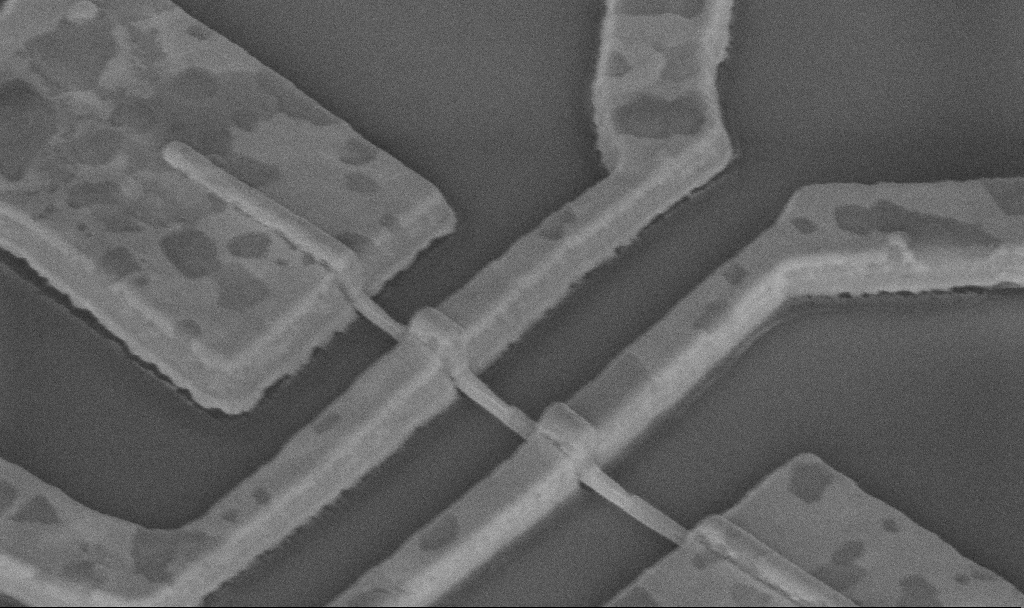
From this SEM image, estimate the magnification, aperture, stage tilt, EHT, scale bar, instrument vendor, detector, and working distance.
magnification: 60 K X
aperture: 30 µm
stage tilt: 45°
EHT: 5 kV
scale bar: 1000 nm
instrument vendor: Zeiss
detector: InLens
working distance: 14.2 mm